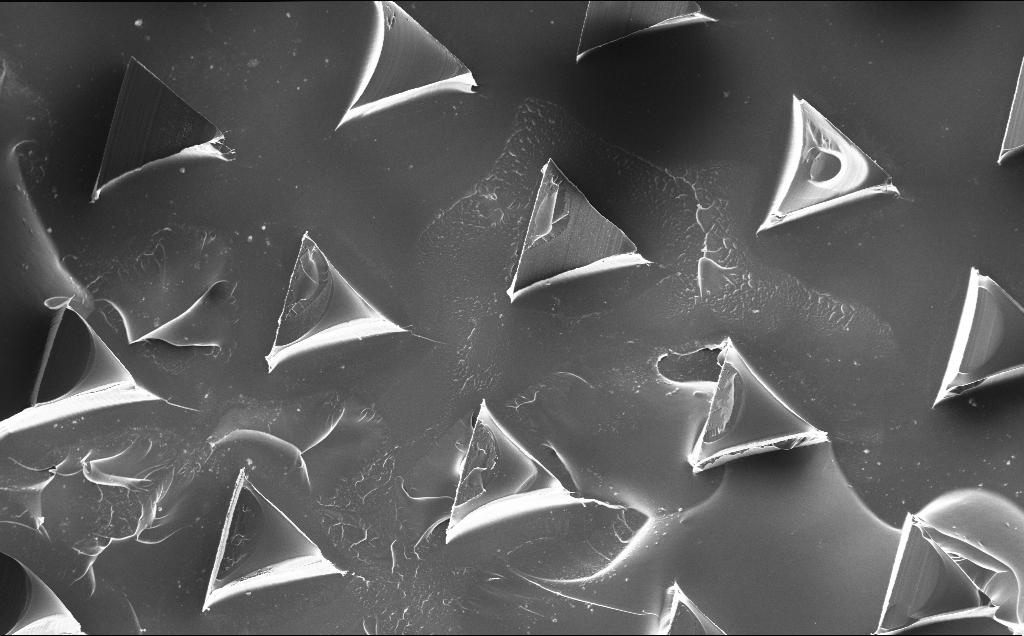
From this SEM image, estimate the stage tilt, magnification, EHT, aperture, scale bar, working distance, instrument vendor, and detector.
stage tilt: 0°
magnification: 0.199 K X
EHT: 10 kV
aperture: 30 µm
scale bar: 200000 nm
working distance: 9 mm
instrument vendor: Zeiss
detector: InLens